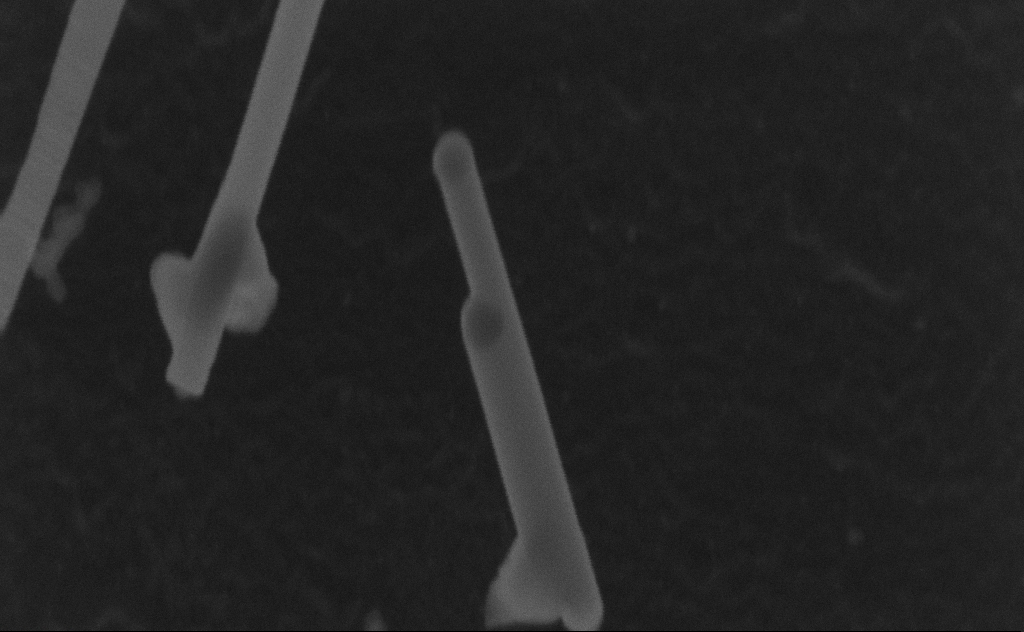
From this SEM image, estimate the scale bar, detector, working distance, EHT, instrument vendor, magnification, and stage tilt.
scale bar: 100 nm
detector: SE2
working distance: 8 mm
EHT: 20 kV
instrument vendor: Zeiss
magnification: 301.66 K X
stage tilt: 0°